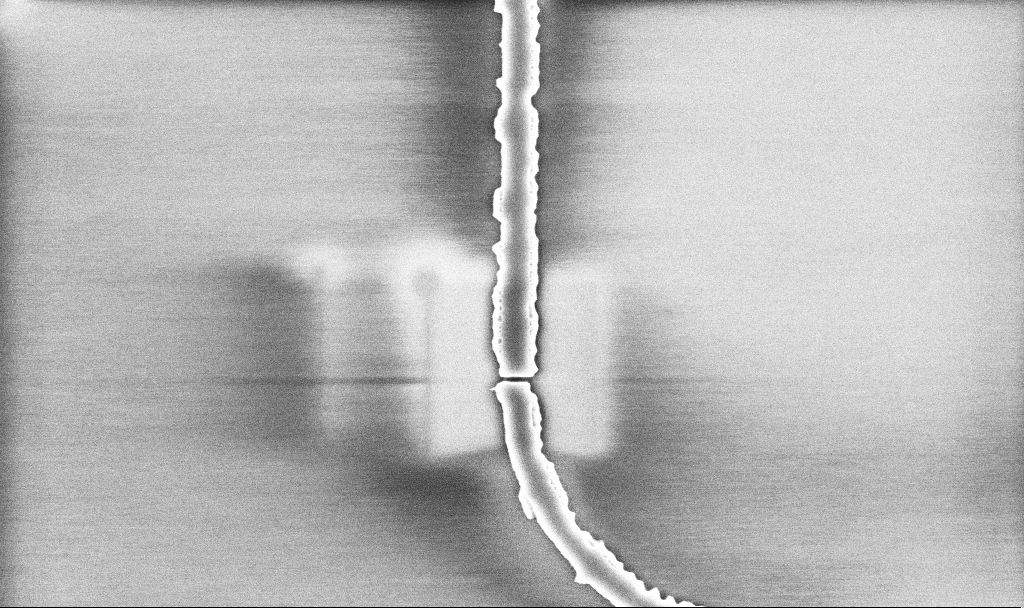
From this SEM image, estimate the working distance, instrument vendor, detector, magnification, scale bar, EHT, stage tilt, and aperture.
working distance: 10.1 mm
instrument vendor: Zeiss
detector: InLens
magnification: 20.67 K X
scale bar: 2000 nm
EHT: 5 kV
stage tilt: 0°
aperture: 30 µm